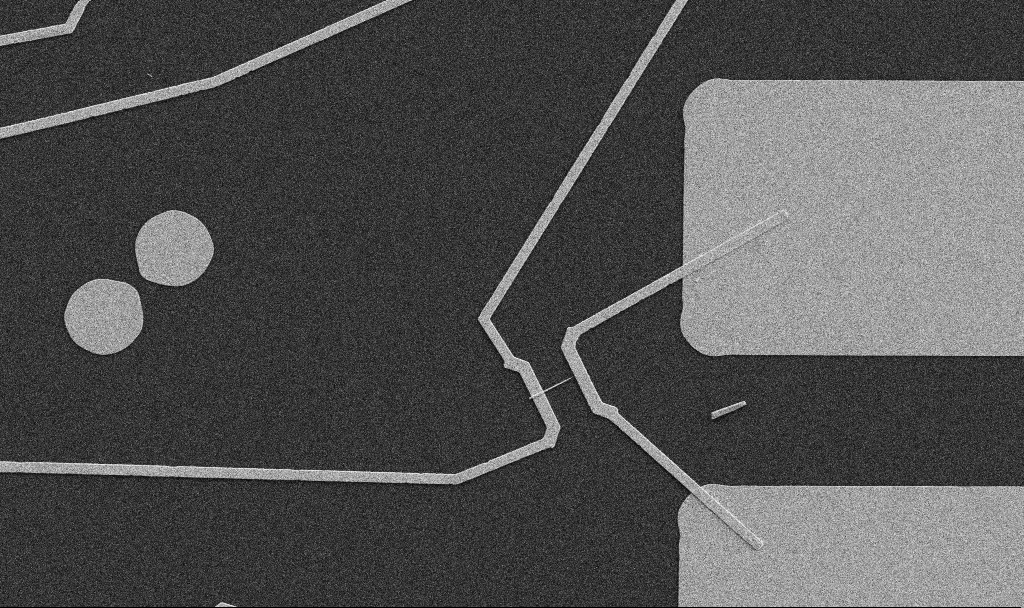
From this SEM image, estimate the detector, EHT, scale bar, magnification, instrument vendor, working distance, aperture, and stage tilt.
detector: SE2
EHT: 5 kV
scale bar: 10000 nm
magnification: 5 K X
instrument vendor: Zeiss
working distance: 10.7 mm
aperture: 30 µm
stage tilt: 0°